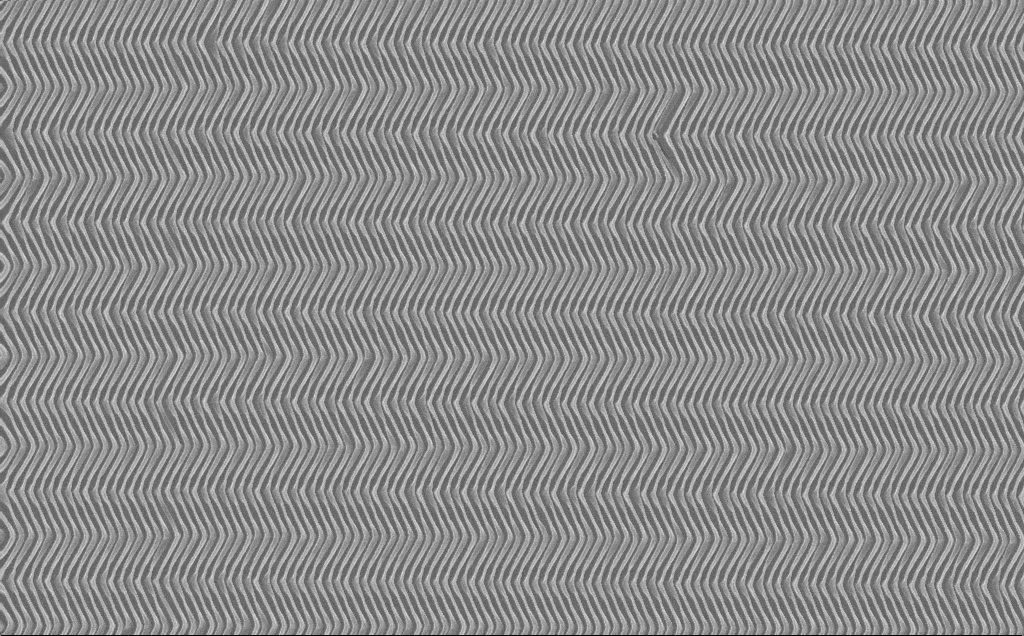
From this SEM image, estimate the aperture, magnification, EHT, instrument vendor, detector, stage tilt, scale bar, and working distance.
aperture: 30 µm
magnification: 16.58 K X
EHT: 10 kV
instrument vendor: Zeiss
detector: InLens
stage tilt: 0°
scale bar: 2000 nm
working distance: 7 mm